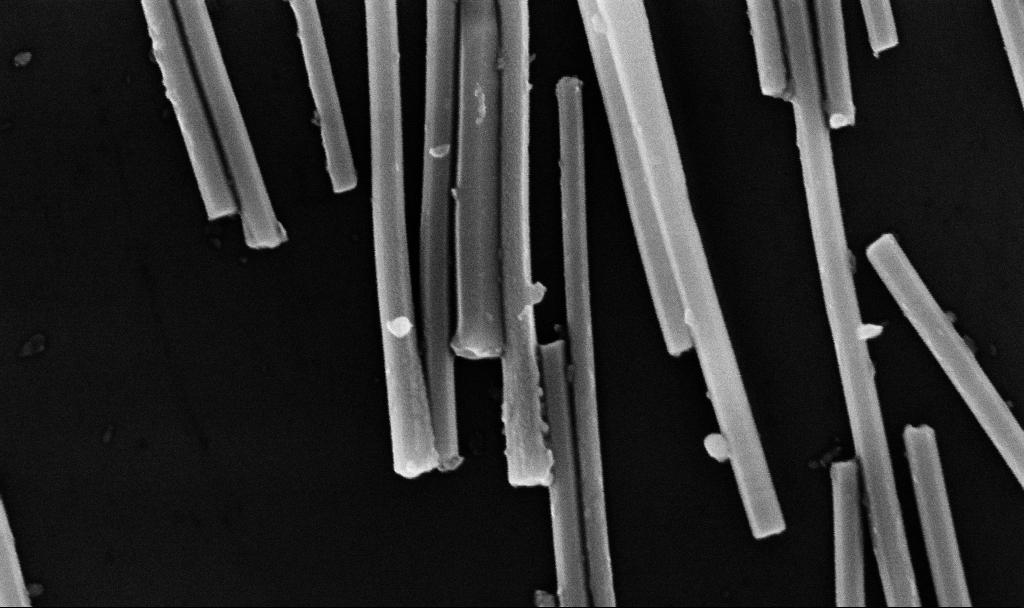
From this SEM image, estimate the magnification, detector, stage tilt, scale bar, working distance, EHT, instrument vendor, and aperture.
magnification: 107.49 K X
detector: InLens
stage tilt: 0°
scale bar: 200 nm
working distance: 8.7 mm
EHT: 10 kV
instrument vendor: Zeiss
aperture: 30 µm